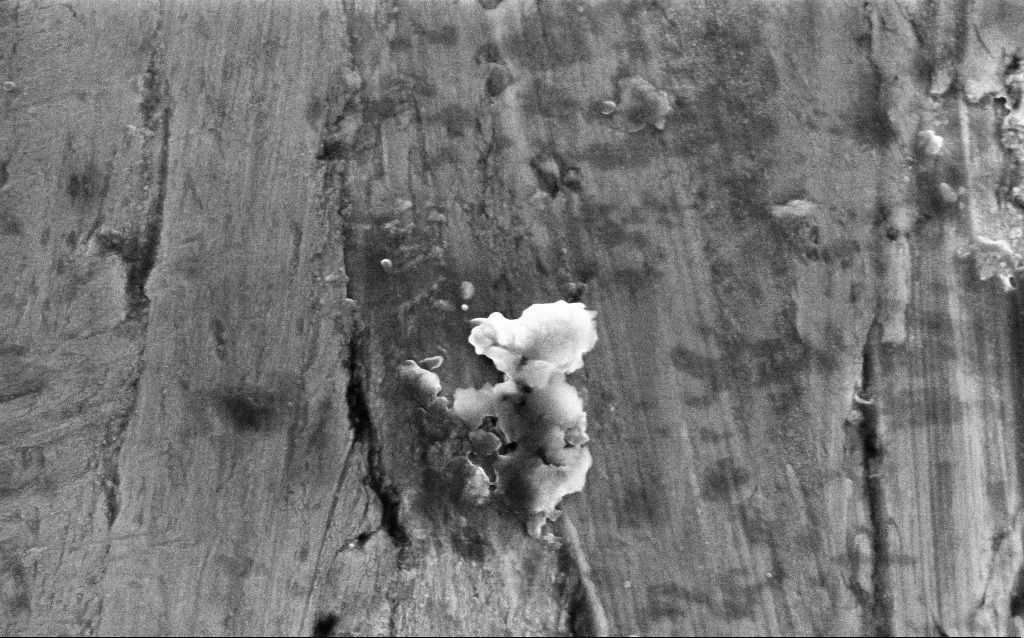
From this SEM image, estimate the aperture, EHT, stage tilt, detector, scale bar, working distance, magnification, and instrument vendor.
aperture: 30 µm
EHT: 5 kV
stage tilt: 18.1°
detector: InLens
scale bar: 200 nm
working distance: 4.3 mm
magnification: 86.12 K X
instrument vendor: Zeiss